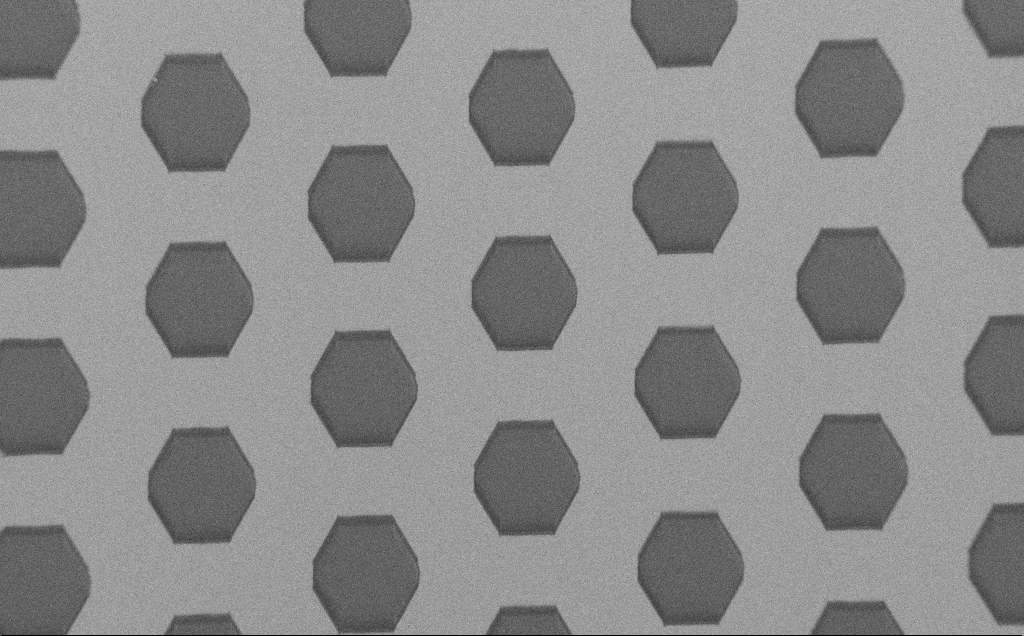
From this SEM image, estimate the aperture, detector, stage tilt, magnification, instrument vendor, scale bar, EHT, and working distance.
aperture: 30 µm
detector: SE2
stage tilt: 0°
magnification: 0.342 K X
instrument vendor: Zeiss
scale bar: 200000 nm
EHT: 1.5 kV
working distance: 6 mm